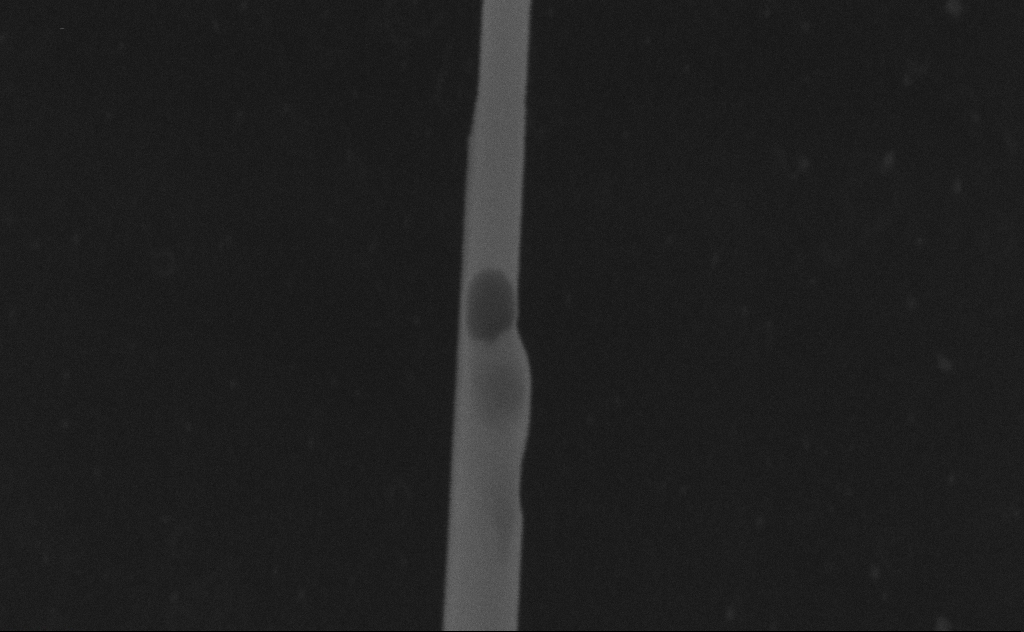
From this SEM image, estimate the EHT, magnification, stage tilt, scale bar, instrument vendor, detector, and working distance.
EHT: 20 kV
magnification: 269.05 K X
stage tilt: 0°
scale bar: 200 nm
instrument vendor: Zeiss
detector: SE2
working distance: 8 mm